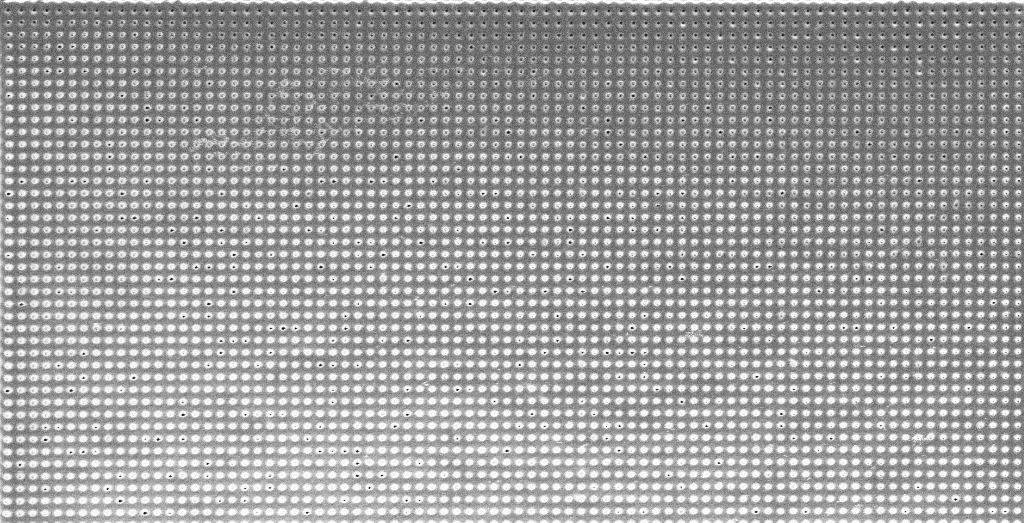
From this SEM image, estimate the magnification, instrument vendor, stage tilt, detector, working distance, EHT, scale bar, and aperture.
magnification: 4.36 K X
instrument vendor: Zeiss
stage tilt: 0°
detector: SE2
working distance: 6.7 mm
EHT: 1.5 kV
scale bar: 10000 nm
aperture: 30 µm